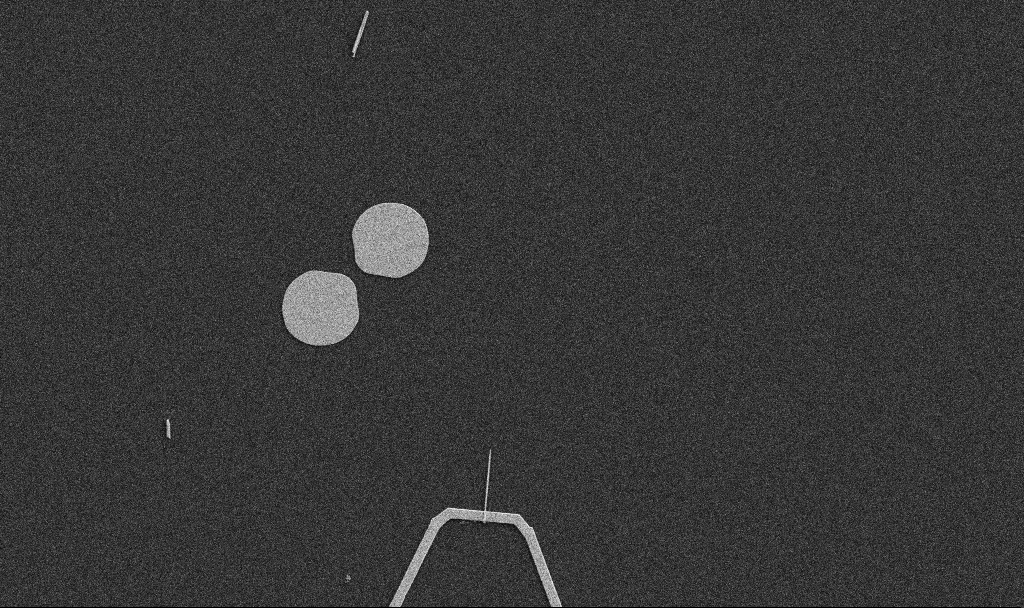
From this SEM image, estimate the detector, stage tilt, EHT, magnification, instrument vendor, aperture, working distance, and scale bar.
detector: SE2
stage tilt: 0°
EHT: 5 kV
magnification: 5 K X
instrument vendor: Zeiss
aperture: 30 µm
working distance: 10.7 mm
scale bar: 10000 nm